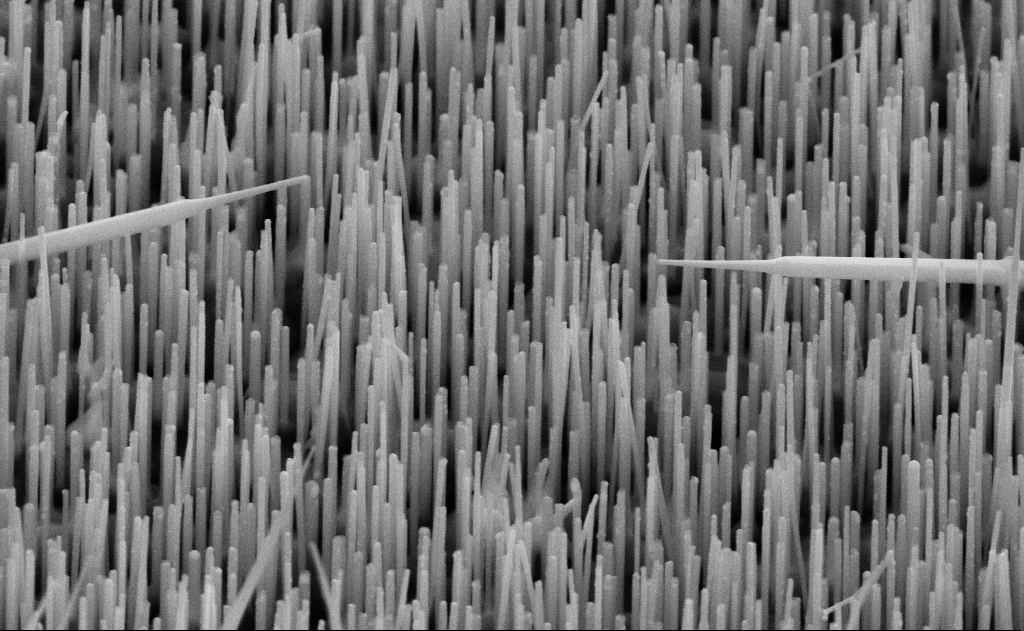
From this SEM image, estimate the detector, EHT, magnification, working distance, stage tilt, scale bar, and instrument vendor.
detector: SE2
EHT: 10 kV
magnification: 60 K X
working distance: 11 mm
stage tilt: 45°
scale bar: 1000 nm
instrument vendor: Zeiss